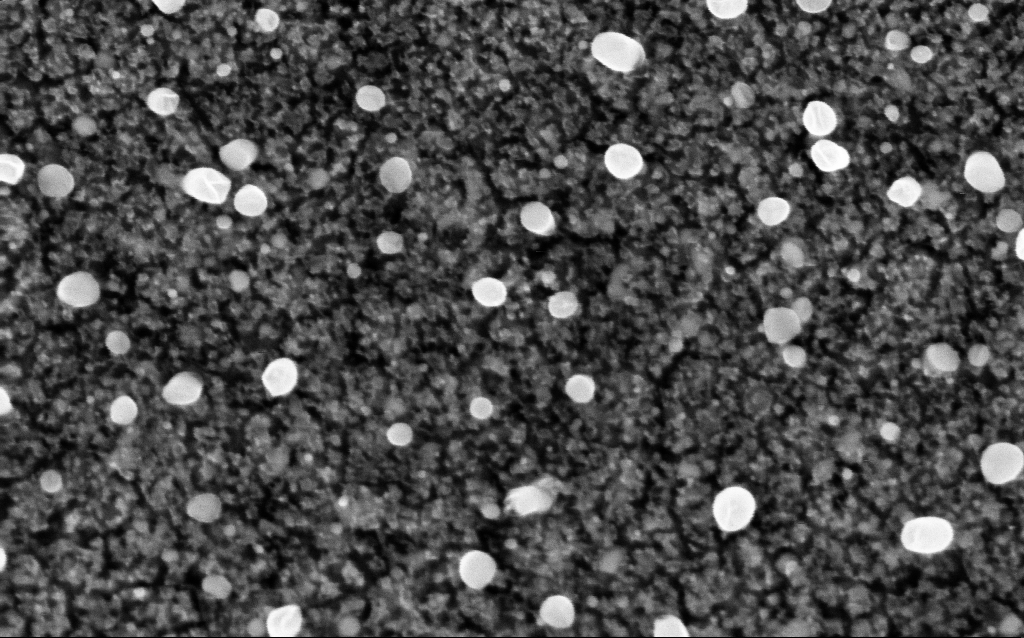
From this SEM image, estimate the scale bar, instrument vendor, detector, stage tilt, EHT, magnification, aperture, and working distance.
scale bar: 100 nm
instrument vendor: Zeiss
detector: InLens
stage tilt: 0°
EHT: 5 kV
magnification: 200 K X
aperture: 30 µm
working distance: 2.1 mm